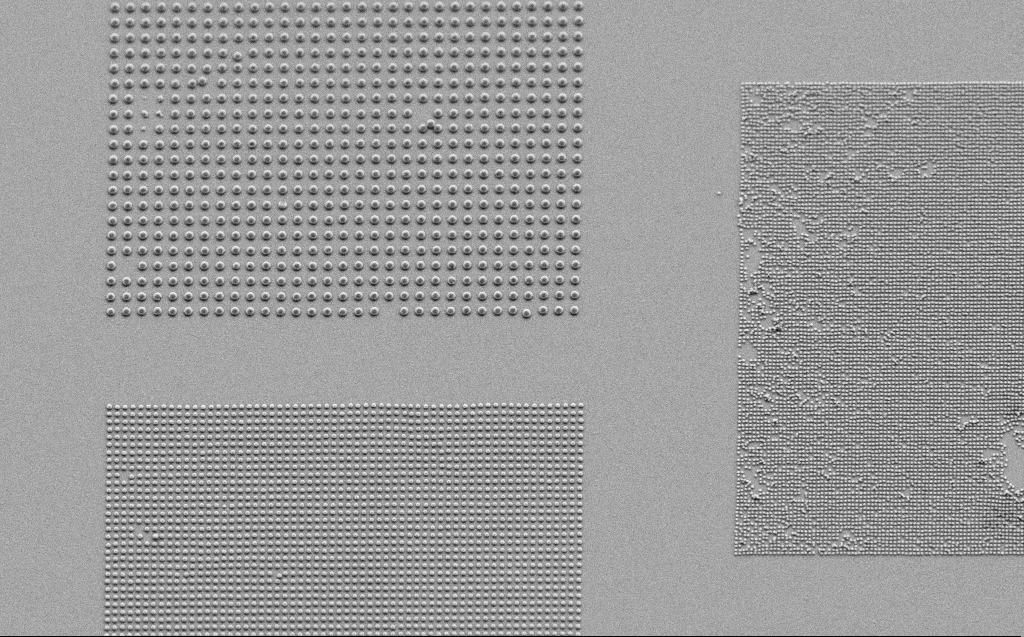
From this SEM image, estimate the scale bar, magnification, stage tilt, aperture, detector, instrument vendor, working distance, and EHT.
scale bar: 10000 nm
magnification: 7.15 K X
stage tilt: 30°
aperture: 30 µm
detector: SE2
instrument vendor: Zeiss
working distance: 5 mm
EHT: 2.5 kV